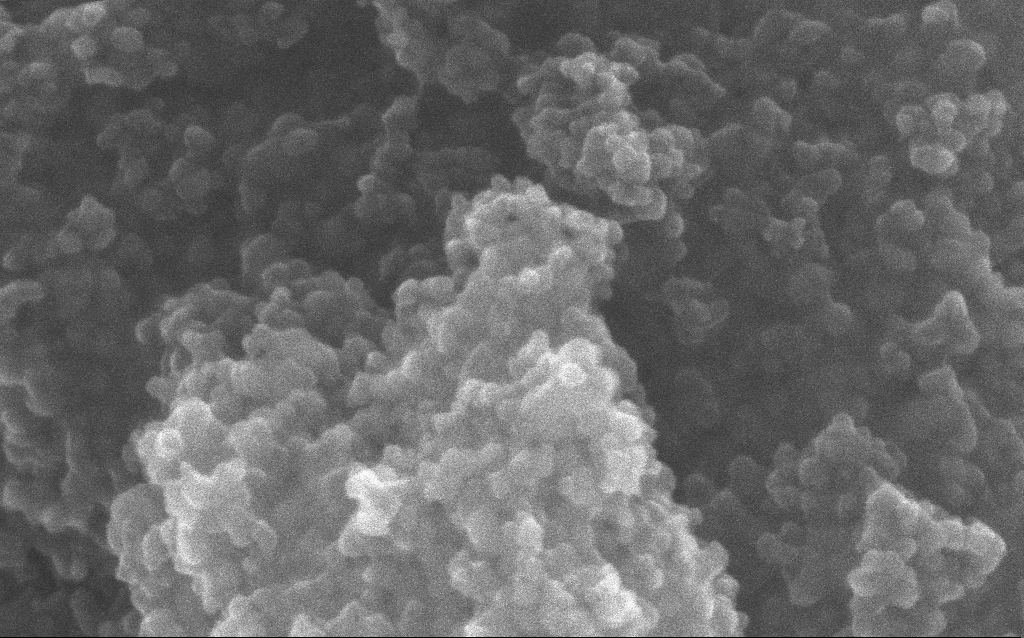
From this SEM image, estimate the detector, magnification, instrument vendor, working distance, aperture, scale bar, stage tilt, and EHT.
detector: InLens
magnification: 348.1 K X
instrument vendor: Zeiss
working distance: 2.7 mm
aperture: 30 µm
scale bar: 100 nm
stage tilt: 0°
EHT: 10 kV